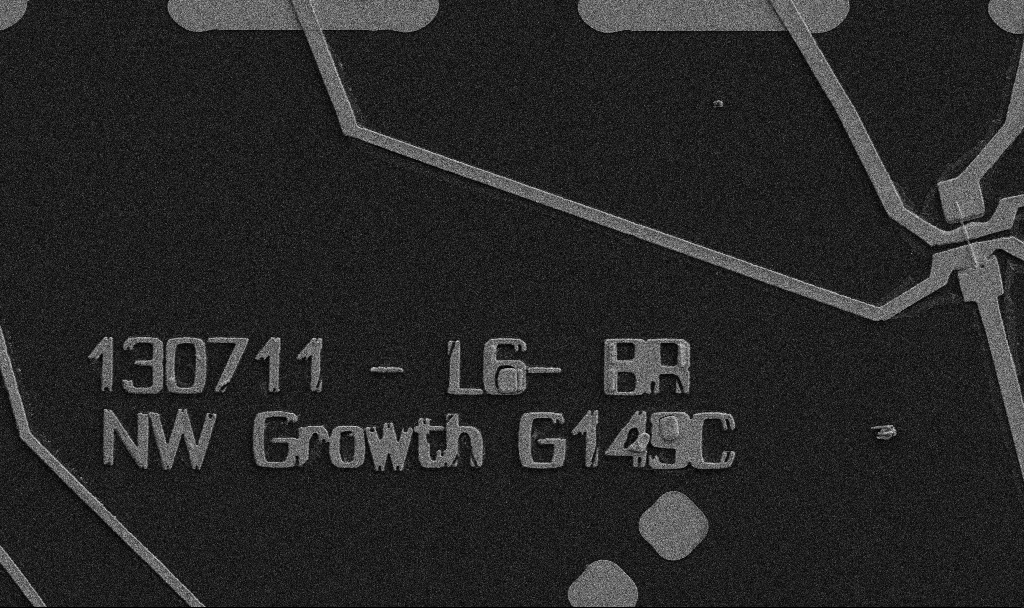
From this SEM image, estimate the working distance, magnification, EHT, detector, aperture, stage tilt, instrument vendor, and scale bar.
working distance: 10.7 mm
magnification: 5 K X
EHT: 5 kV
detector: SE2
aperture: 30 µm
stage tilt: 0°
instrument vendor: Zeiss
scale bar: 10000 nm